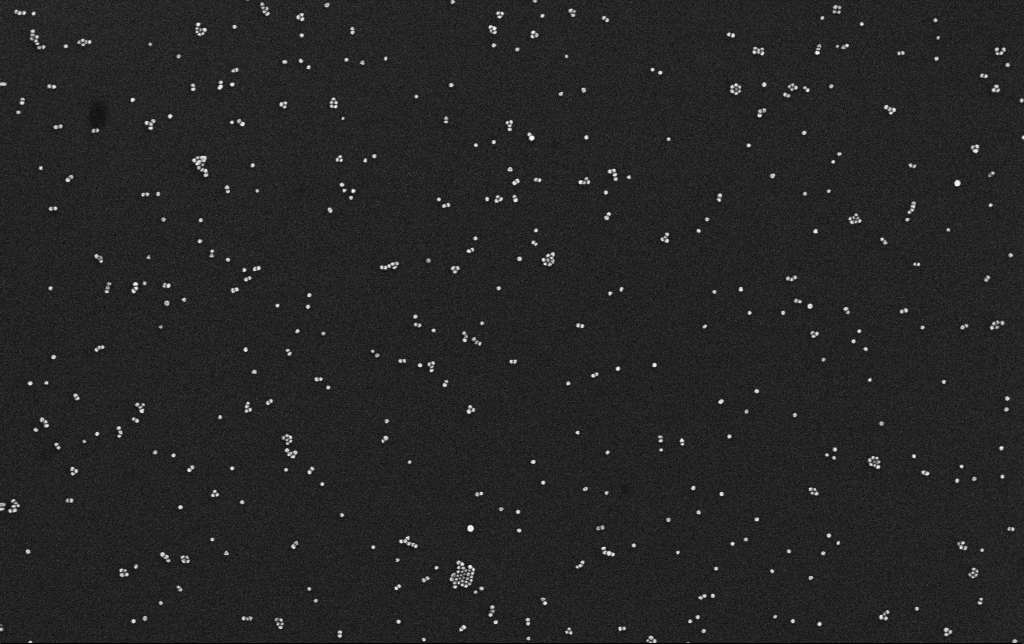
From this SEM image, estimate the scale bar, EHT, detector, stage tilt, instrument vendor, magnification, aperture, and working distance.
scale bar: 200 nm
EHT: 10 kV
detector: InLens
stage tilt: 0°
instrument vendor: Zeiss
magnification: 100 K X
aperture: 30 µm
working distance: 3.1 mm